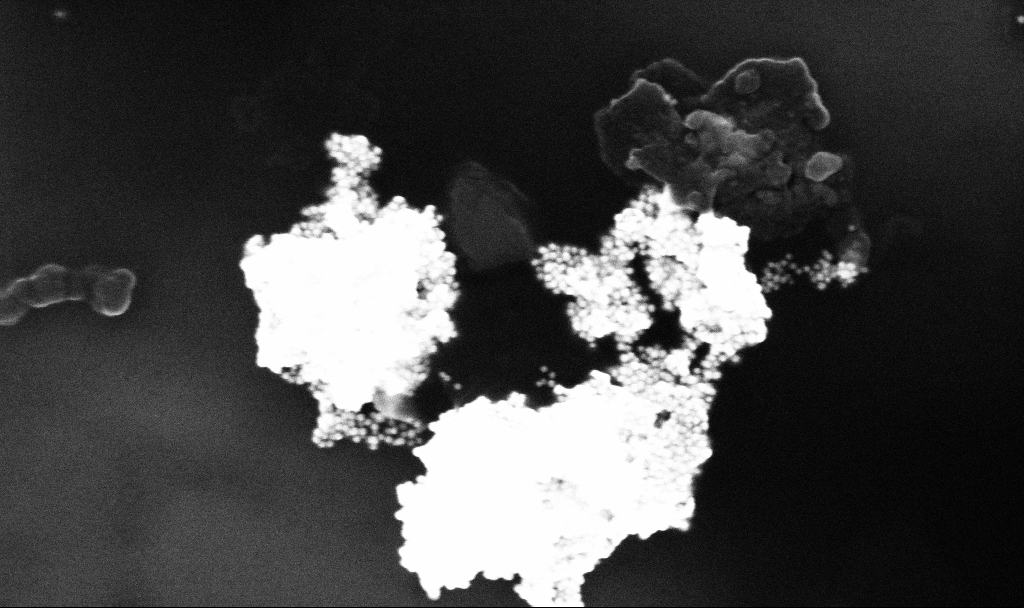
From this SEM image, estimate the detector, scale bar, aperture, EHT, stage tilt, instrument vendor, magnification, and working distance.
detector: InLens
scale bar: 100 nm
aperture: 30 µm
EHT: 10 kV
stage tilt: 0°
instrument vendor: Zeiss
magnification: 133.41 K X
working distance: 3.4 mm